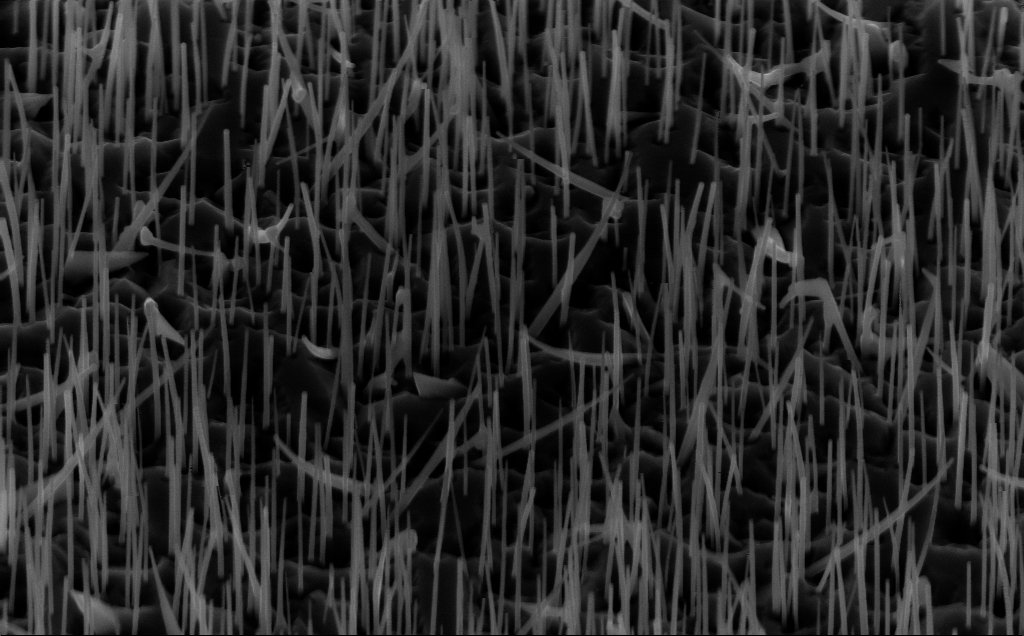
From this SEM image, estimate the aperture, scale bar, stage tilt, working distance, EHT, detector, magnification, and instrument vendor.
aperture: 30 µm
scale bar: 1000 nm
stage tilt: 45°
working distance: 5 mm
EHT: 10 kV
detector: InLens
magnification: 40 K X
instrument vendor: Zeiss